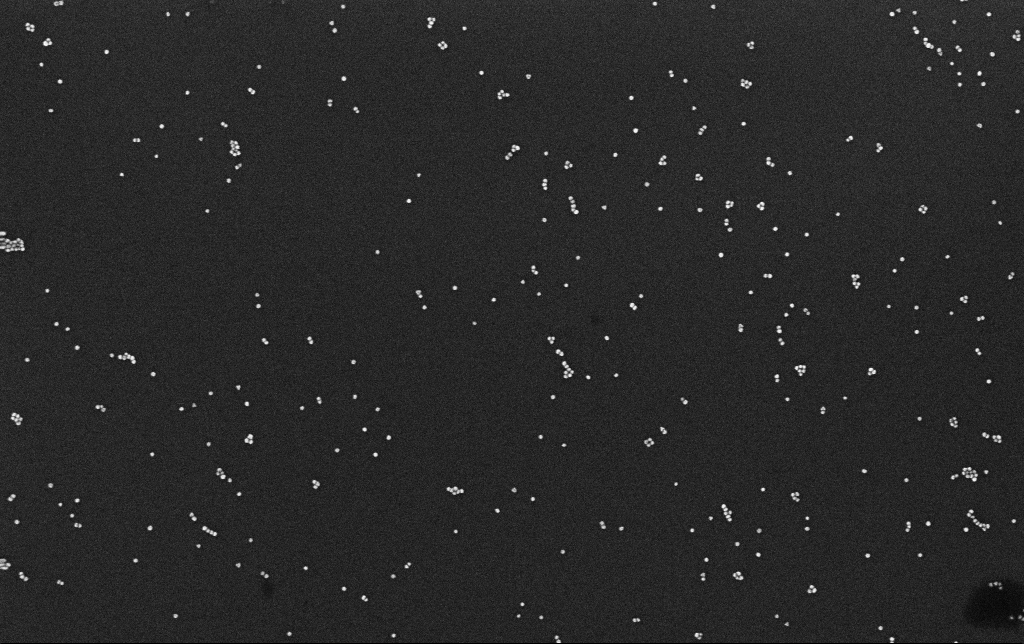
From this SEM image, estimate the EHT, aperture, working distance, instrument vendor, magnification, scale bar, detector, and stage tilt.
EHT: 10 kV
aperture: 30 µm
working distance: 3.1 mm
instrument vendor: Zeiss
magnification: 100 K X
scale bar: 200 nm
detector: InLens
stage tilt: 0°